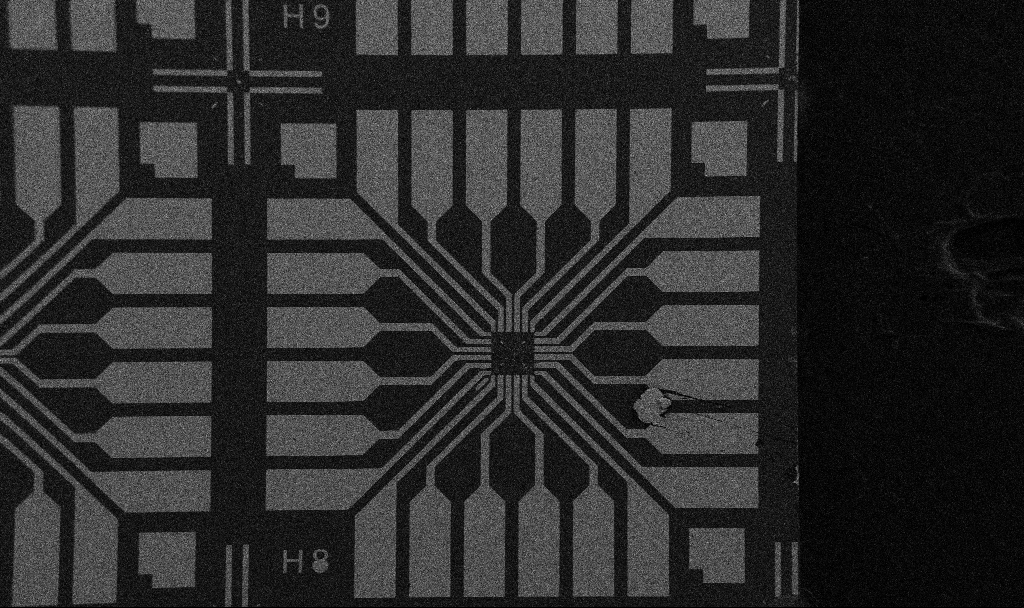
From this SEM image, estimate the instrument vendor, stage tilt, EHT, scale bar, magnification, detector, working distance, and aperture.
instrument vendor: Zeiss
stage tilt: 0°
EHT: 5 kV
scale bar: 200000 nm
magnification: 0.1 K X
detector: SE2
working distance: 10.7 mm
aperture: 30 µm